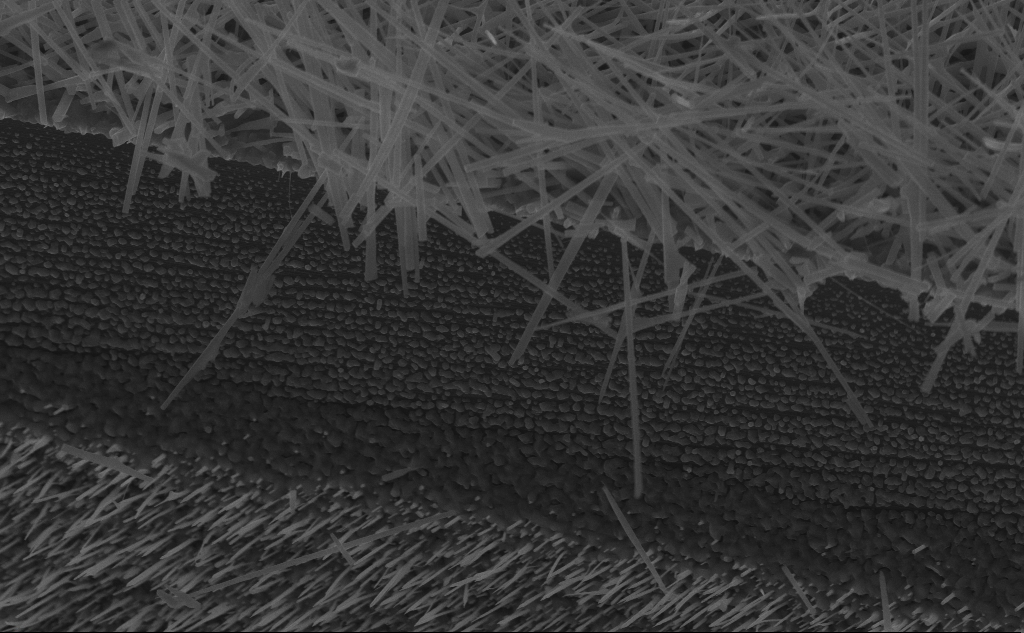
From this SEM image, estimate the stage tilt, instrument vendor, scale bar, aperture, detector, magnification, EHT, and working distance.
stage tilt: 45°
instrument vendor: Zeiss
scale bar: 2000 nm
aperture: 30 µm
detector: InLens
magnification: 23.08 K X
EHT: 10 kV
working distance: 5 mm